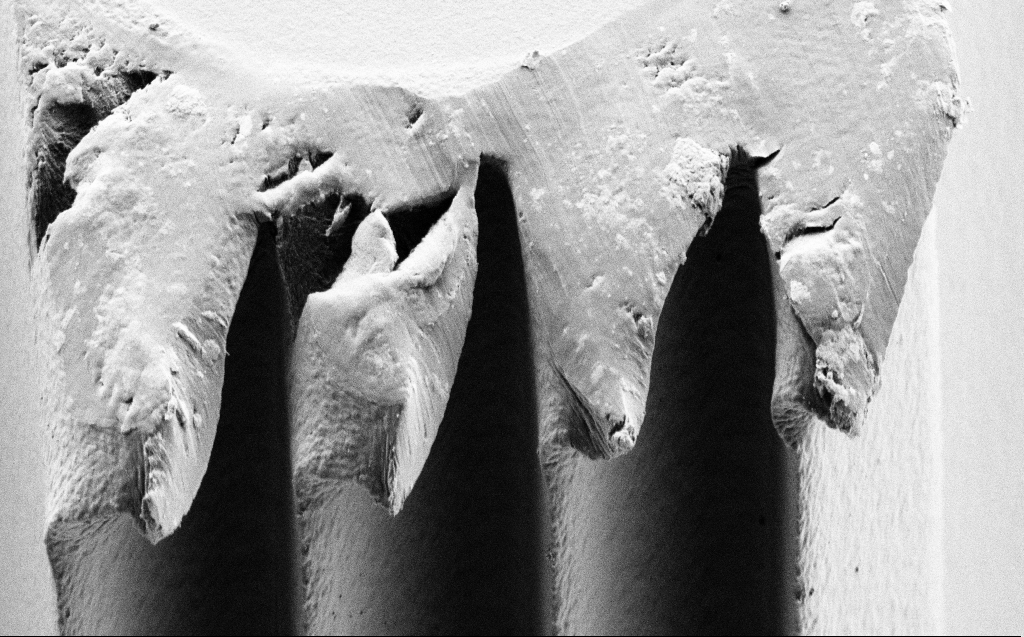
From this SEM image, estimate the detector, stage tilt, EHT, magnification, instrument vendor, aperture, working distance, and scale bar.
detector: SE2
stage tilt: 44.6°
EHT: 1 kV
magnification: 7.86 K X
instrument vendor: Zeiss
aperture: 30 µm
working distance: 5 mm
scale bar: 2000 nm